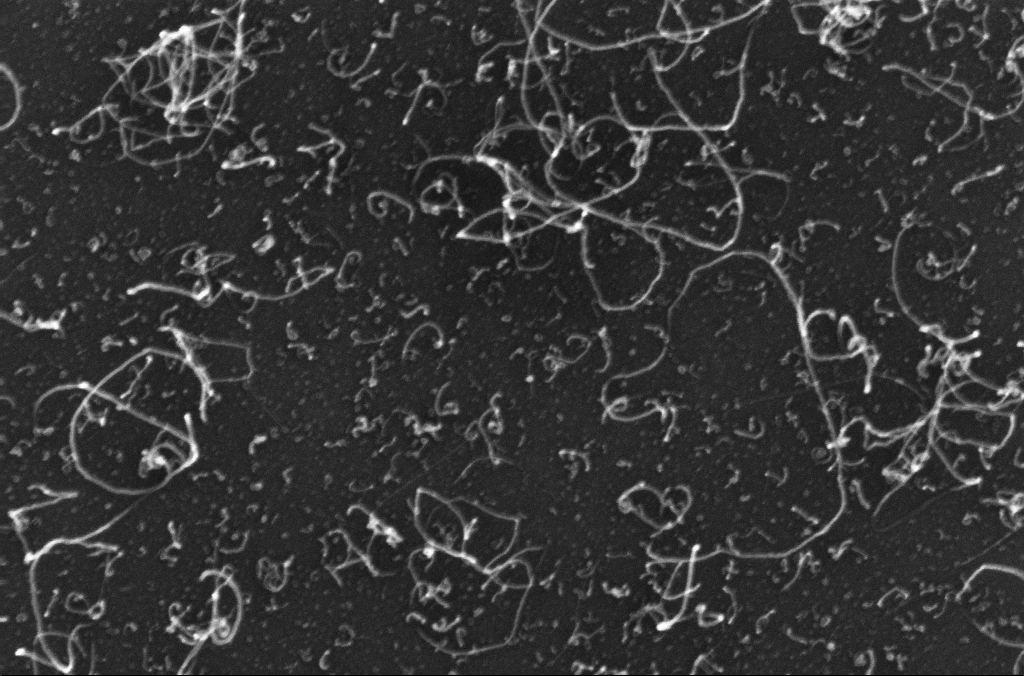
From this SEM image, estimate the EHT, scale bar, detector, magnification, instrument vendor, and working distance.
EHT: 10 kV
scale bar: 100 nm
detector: InLens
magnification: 150 K X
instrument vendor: Zeiss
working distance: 3.3 mm